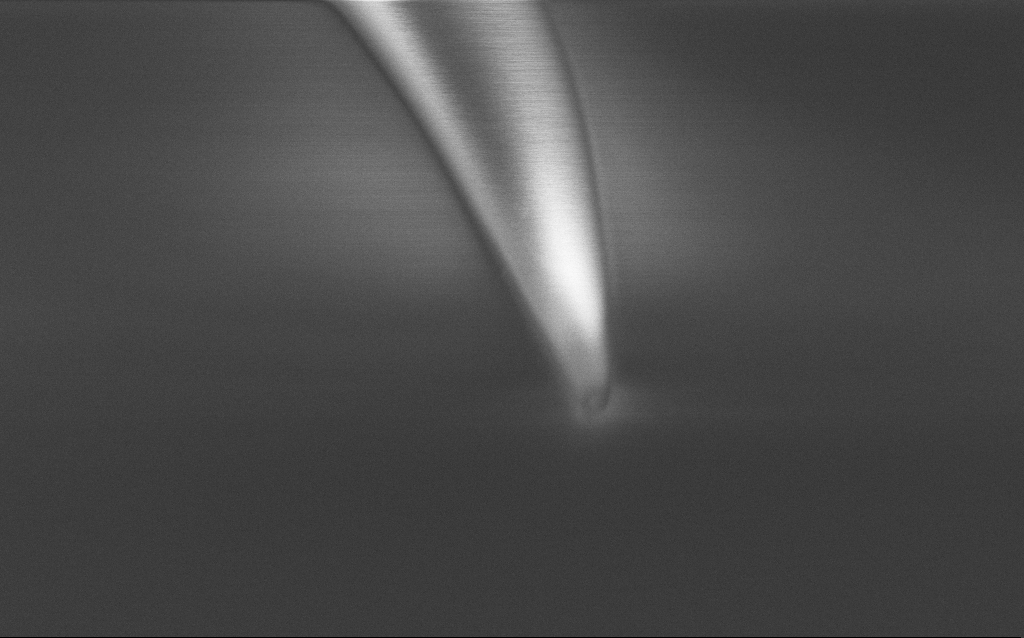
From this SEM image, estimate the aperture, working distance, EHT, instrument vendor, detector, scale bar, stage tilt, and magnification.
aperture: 30 µm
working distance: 7 mm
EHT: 1 kV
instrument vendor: Zeiss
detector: InLens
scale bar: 200 nm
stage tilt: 45°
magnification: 100 K X